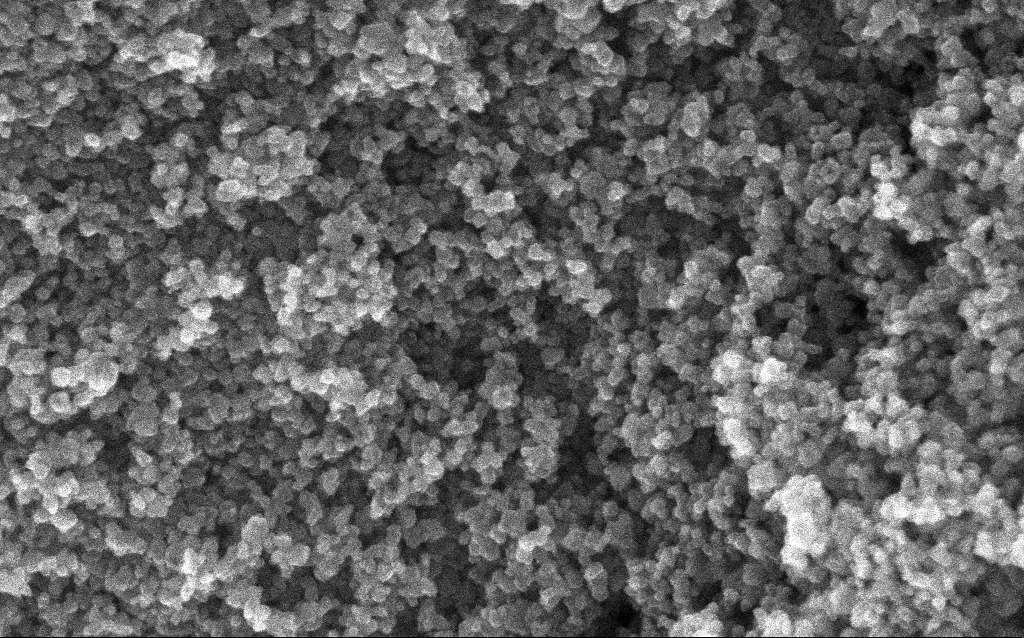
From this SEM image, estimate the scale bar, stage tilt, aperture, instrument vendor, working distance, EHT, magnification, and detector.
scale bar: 100 nm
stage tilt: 0°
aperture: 30 µm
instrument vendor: Zeiss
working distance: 2.6 mm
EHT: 10 kV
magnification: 211.33 K X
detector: InLens